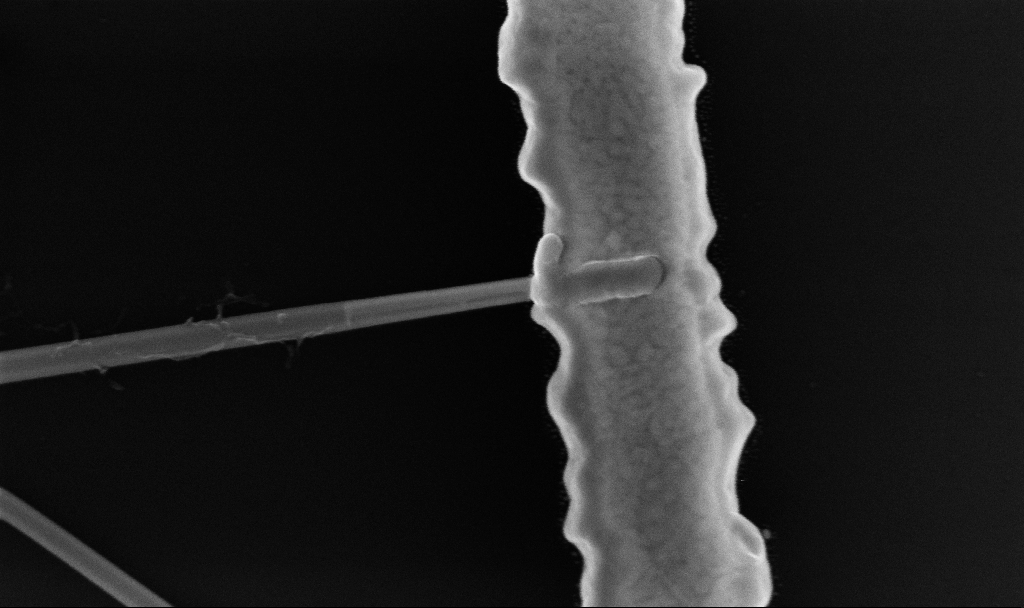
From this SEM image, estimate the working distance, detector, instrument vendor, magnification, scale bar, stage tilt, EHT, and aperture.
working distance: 6.7 mm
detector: InLens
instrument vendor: Zeiss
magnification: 139.2 K X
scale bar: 200 nm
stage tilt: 0°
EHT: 10 kV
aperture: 30 µm